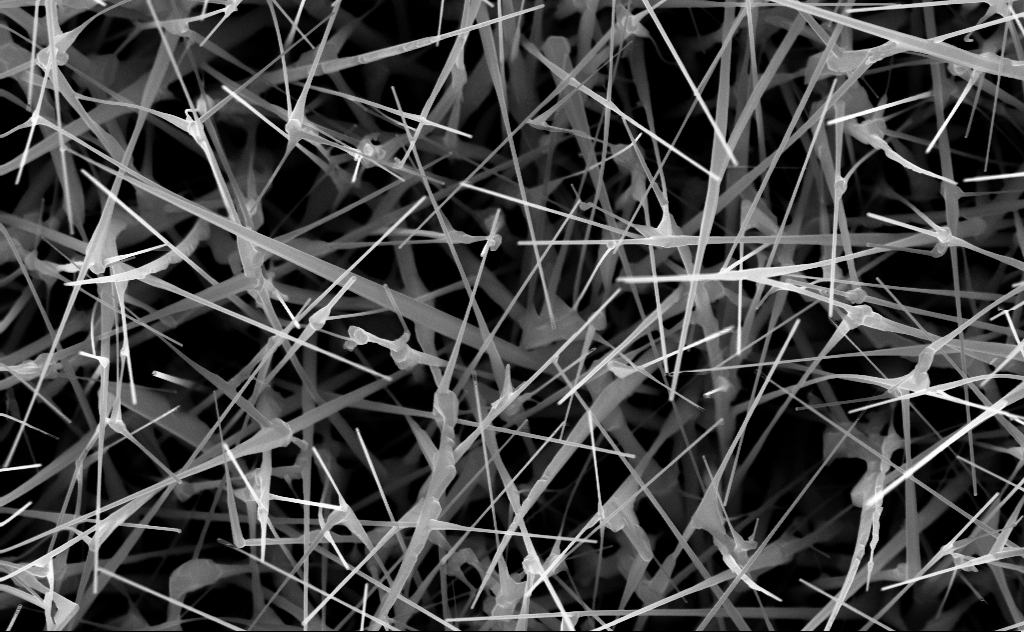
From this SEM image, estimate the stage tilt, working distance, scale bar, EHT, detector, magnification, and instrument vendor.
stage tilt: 0°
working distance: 6 mm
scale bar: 1000 nm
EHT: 10 kV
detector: InLens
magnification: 40 K X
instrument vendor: Zeiss